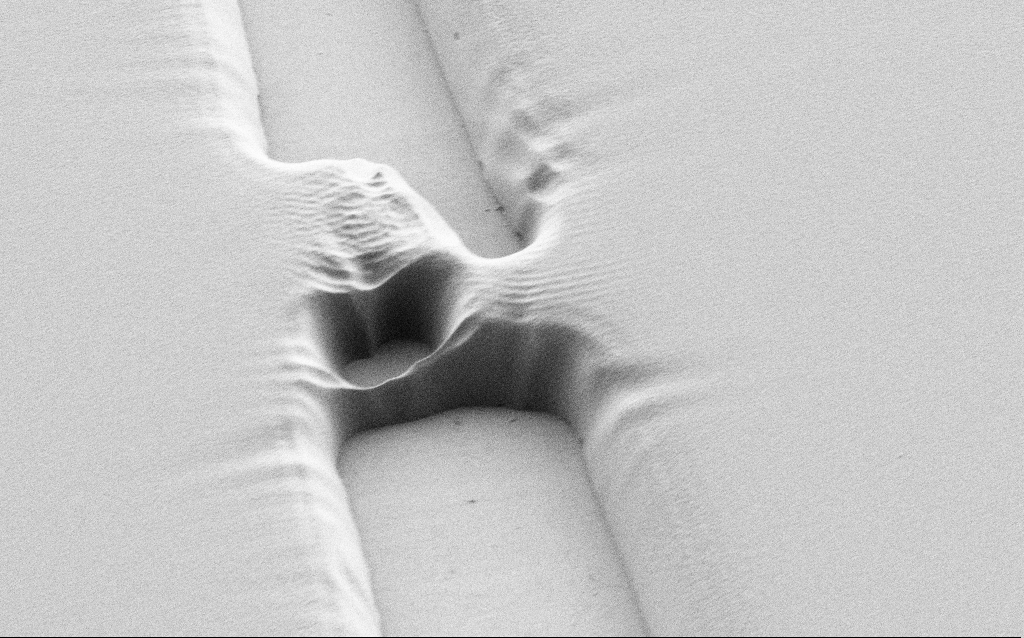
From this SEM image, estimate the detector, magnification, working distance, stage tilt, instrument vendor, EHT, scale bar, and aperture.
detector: SE2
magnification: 6.51 K X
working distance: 5 mm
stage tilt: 36°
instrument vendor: Zeiss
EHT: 1 kV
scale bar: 10000 nm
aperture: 30 µm